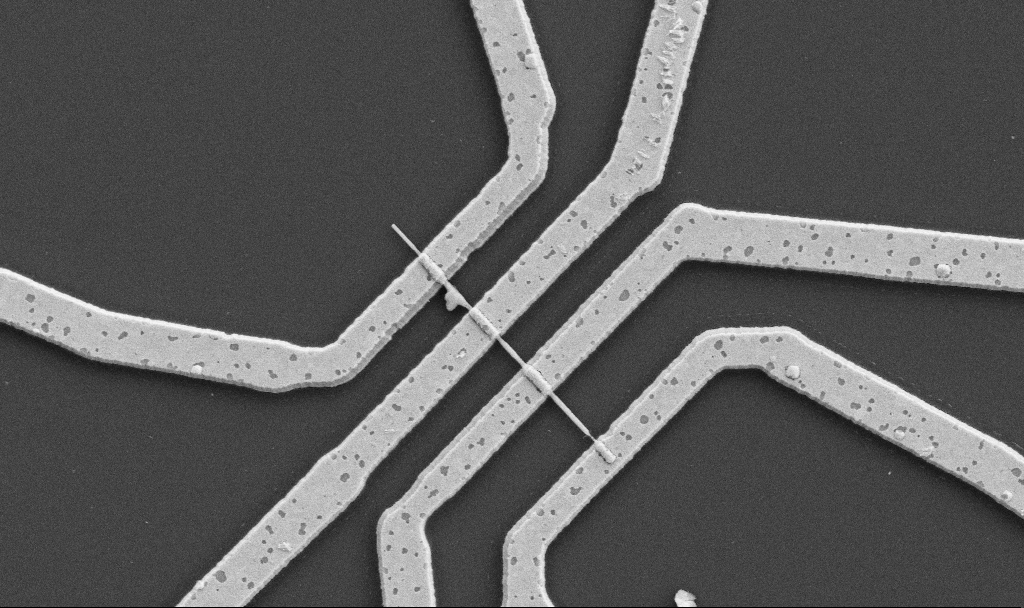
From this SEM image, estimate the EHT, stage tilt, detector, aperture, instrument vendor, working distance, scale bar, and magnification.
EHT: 5 kV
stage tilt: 0°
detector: SE2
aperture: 30 µm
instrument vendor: Zeiss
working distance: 10.6 mm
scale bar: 1000 nm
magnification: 20 K X